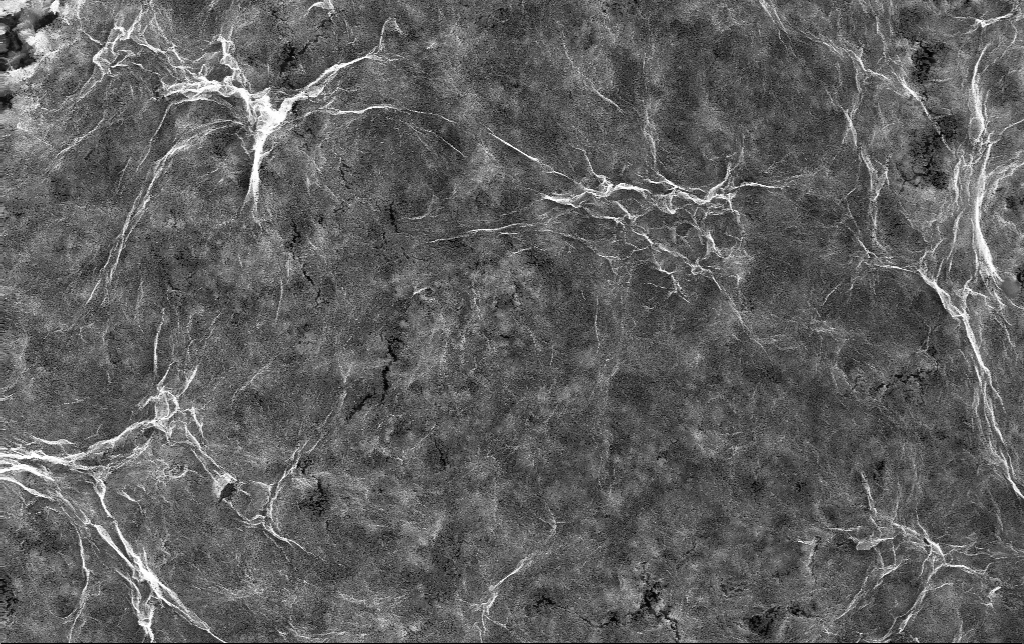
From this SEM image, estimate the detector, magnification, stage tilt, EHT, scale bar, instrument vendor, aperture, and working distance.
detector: InLens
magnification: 20 K X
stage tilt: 0°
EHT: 10 kV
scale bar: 2000 nm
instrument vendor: Zeiss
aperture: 30 µm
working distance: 3 mm